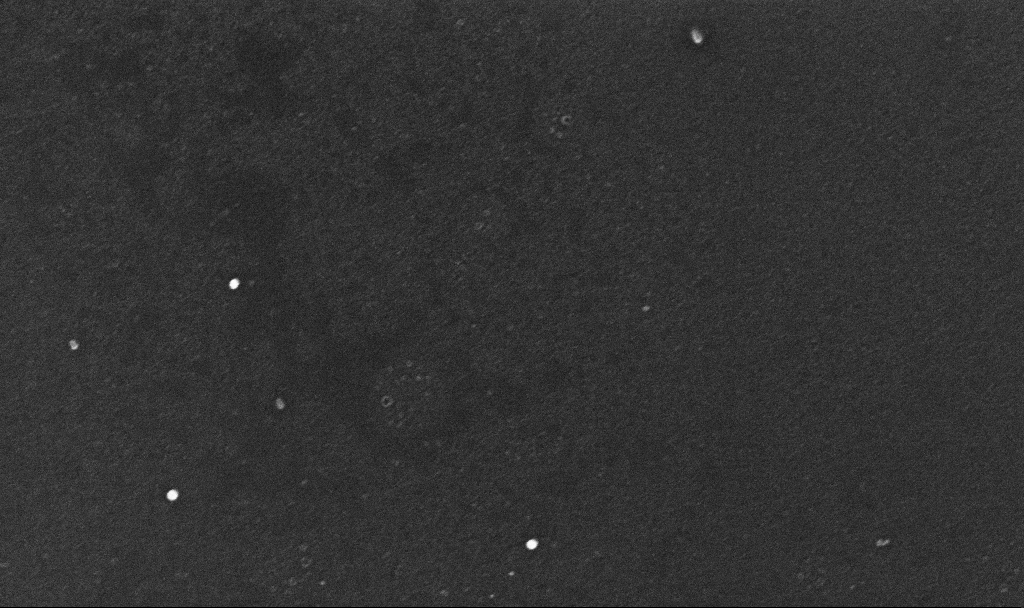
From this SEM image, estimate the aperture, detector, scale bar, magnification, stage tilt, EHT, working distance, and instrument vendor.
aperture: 30 µm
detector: InLens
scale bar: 200 nm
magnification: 150 K X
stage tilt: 0°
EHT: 10 kV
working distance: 3 mm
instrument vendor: Zeiss